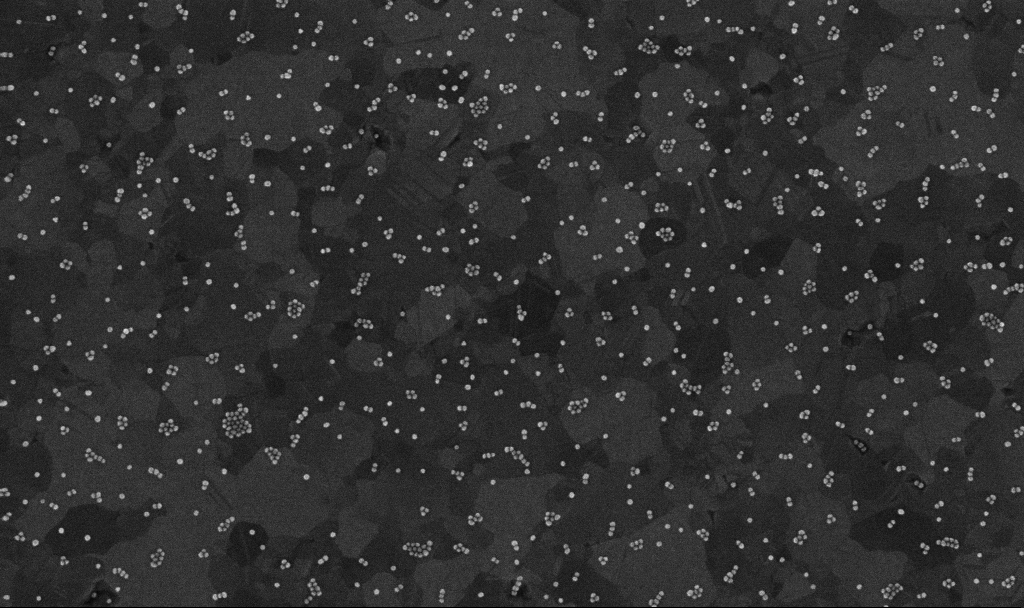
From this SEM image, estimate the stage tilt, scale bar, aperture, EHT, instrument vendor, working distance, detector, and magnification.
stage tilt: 0°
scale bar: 200 nm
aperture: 30 µm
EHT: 10 kV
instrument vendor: Zeiss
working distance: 3.4 mm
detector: InLens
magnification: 100 K X